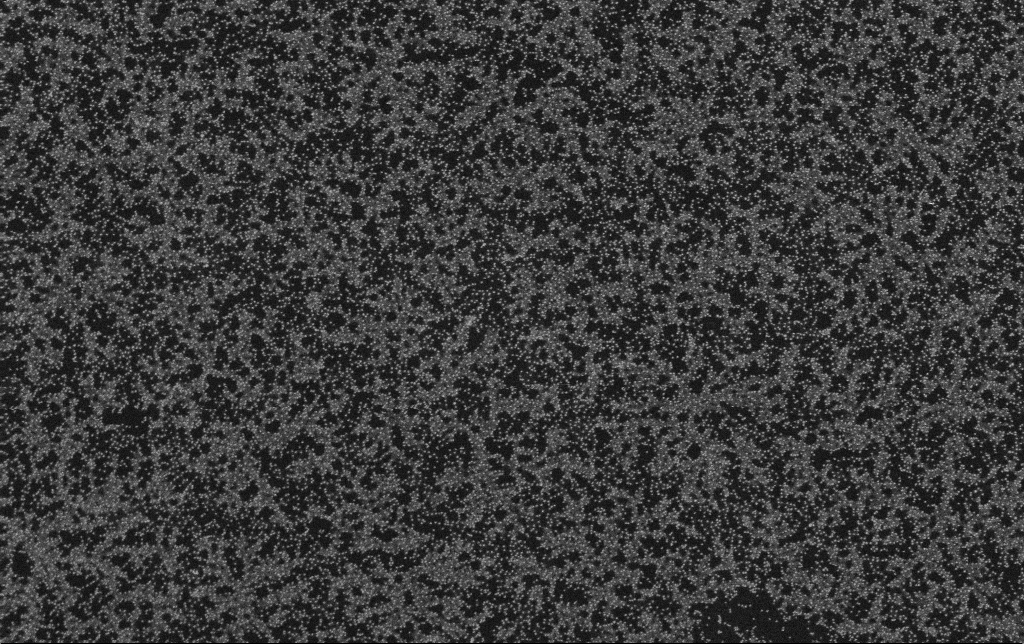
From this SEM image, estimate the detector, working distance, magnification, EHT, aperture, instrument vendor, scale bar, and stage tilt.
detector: SE2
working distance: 11.3 mm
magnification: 50 K X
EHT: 8 kV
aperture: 30 µm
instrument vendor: Zeiss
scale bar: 1000 nm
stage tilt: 0°